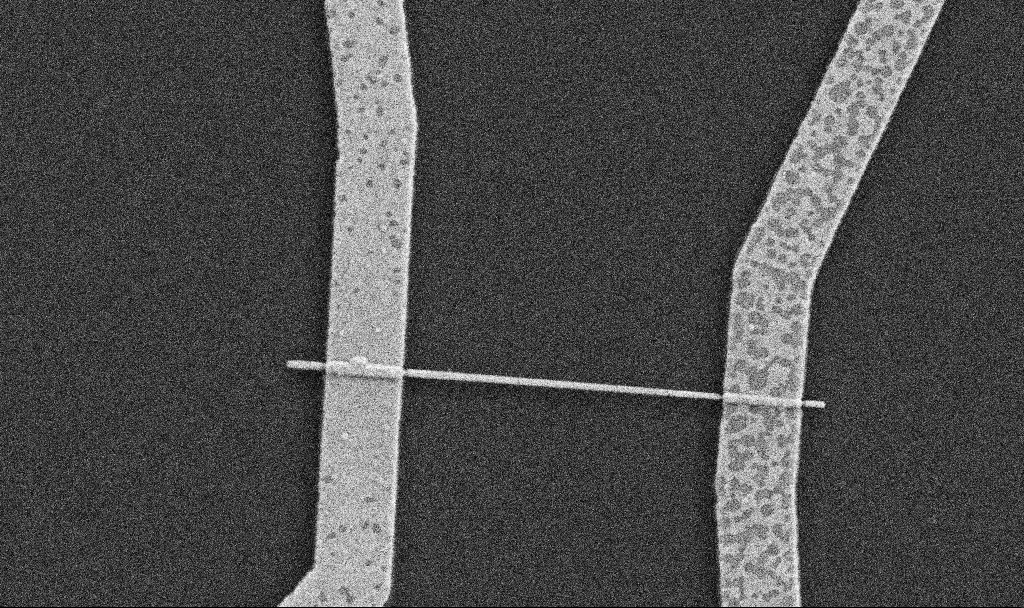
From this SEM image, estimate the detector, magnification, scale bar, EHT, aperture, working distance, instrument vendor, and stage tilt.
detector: SE2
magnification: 30 K X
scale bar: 1000 nm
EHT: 5 kV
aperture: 30 µm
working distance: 8.7 mm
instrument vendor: Zeiss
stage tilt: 0°